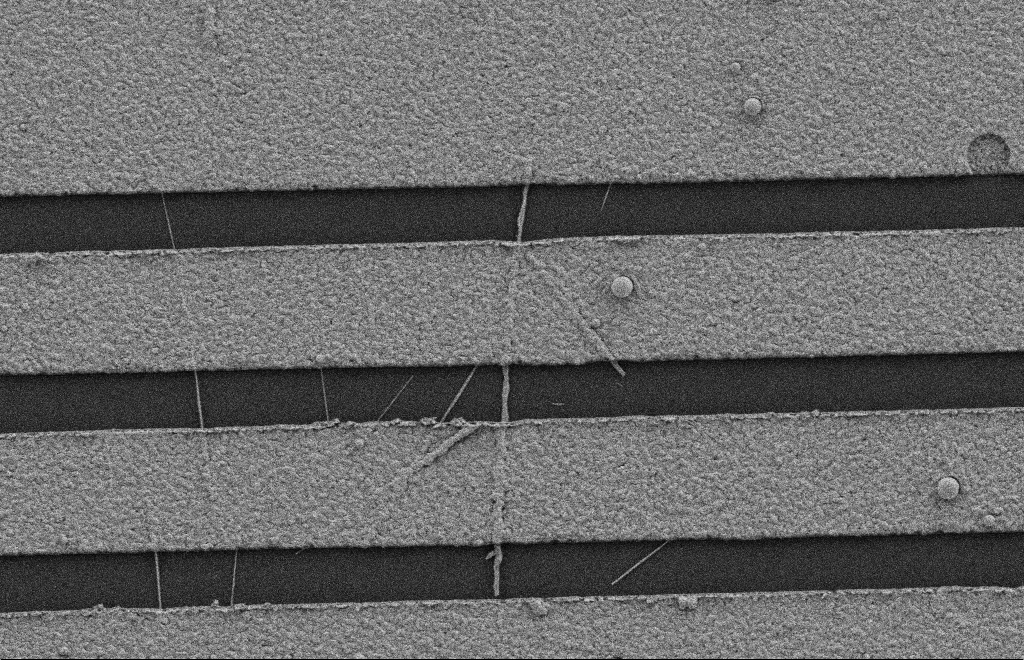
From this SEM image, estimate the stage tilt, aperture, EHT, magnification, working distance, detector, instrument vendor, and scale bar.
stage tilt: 0°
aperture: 20 µm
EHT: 2 kV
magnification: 16.44 K X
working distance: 9 mm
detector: SE2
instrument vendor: Zeiss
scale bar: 2000 nm